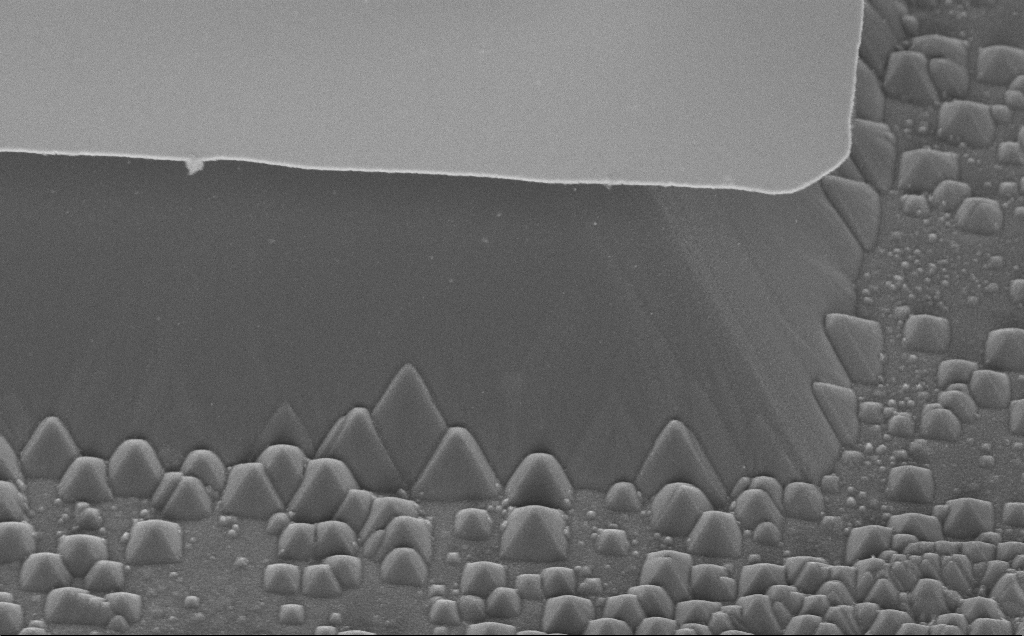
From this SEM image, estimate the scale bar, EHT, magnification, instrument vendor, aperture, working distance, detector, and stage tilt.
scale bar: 2000 nm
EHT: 5 kV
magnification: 11.99 K X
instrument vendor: Zeiss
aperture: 30 µm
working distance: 13 mm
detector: InLens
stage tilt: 45°